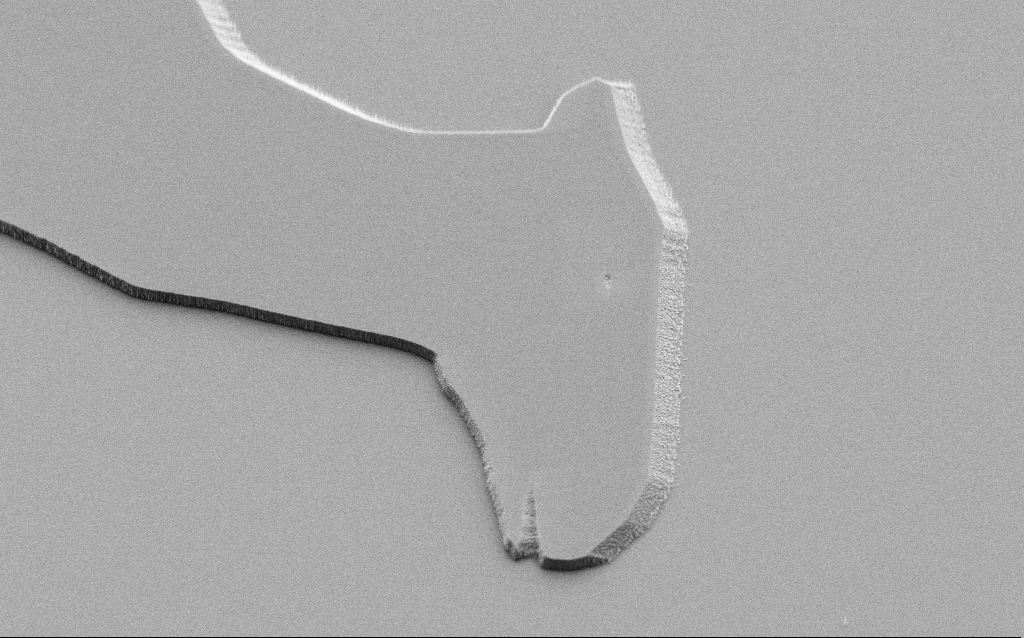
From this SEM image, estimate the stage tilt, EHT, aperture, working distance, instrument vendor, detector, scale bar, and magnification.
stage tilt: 45°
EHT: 3 kV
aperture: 30 µm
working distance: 6 mm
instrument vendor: Zeiss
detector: SE2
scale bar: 10000 nm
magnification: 2.87 K X